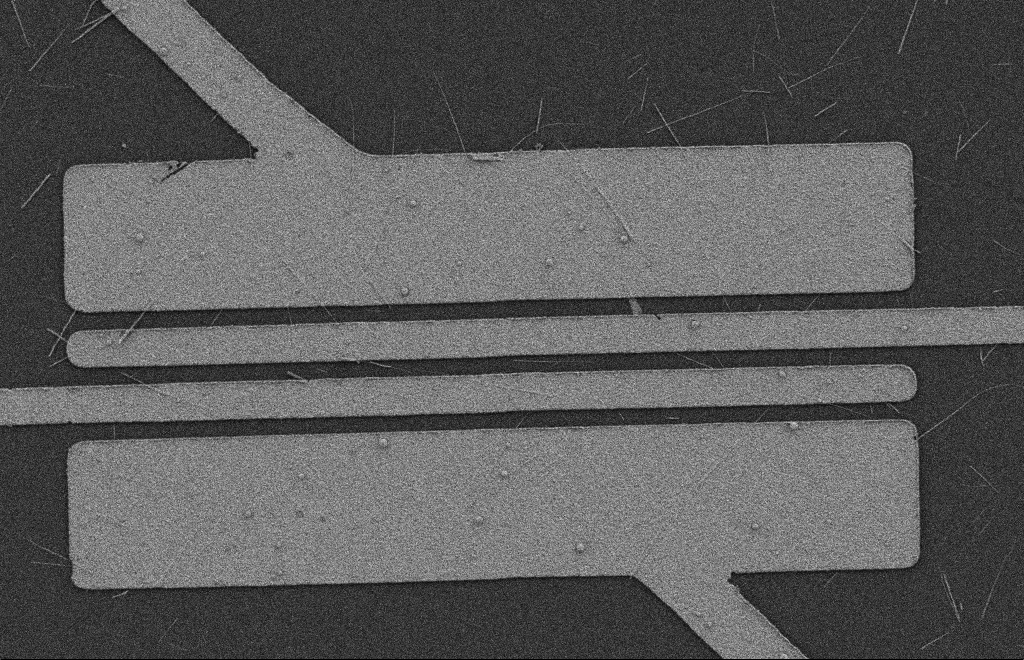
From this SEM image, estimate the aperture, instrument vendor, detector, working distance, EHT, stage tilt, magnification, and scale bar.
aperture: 20 µm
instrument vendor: Zeiss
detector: SE2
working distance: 9 mm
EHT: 2 kV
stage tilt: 0°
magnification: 5.1 K X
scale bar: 2000 nm